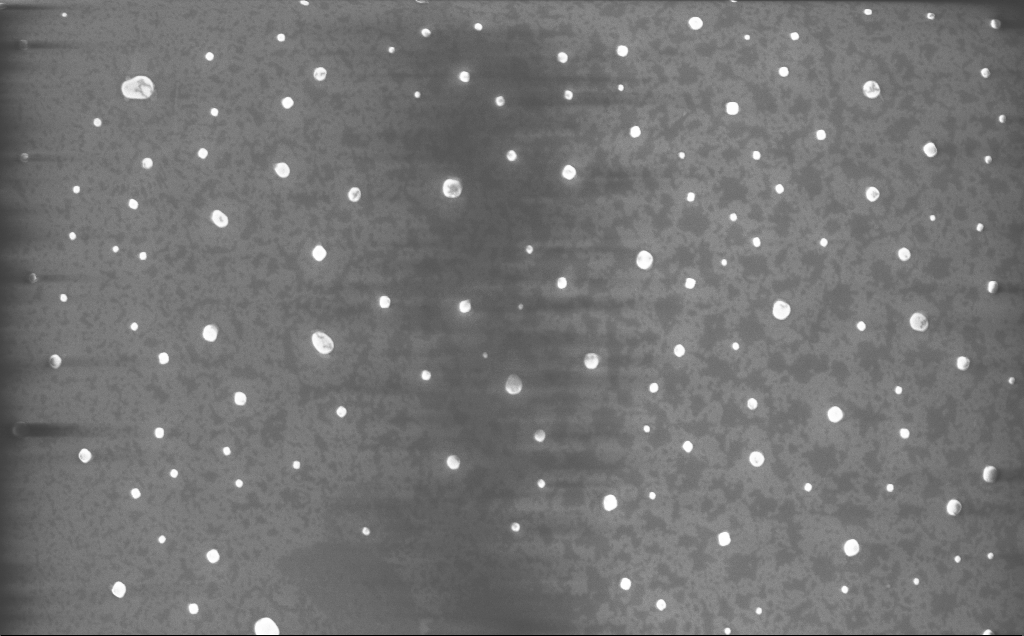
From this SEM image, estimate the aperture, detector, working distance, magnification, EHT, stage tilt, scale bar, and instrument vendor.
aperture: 30 µm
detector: InLens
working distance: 4 mm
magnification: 20.63 K X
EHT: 10 kV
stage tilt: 0°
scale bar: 1000 nm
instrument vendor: Zeiss